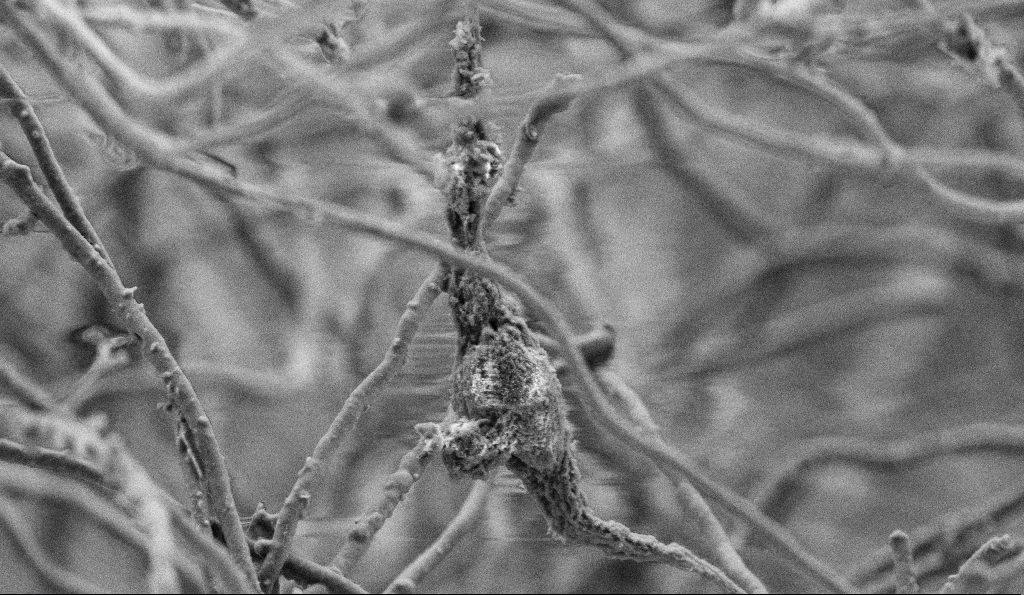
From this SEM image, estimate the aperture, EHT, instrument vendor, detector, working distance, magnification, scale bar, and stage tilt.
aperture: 30 µm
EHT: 3 kV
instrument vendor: Zeiss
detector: SE2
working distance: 4.9 mm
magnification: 10 K X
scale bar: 2000 nm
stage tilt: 0°